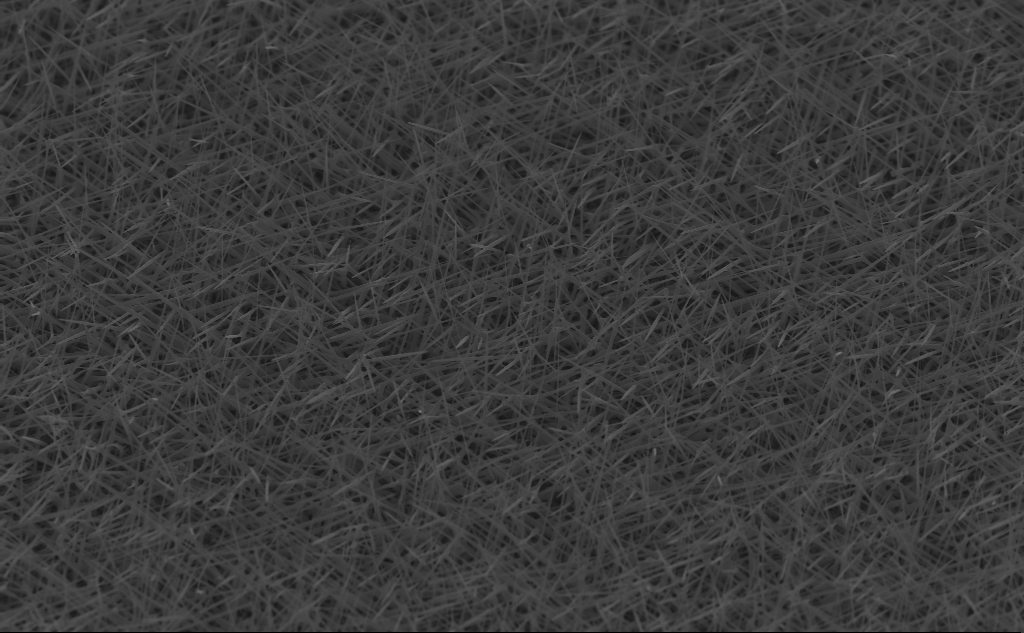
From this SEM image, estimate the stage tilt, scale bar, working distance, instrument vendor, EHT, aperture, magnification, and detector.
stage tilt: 45°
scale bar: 2000 nm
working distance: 5 mm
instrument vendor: Zeiss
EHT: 10 kV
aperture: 30 µm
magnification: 11.77 K X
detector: InLens